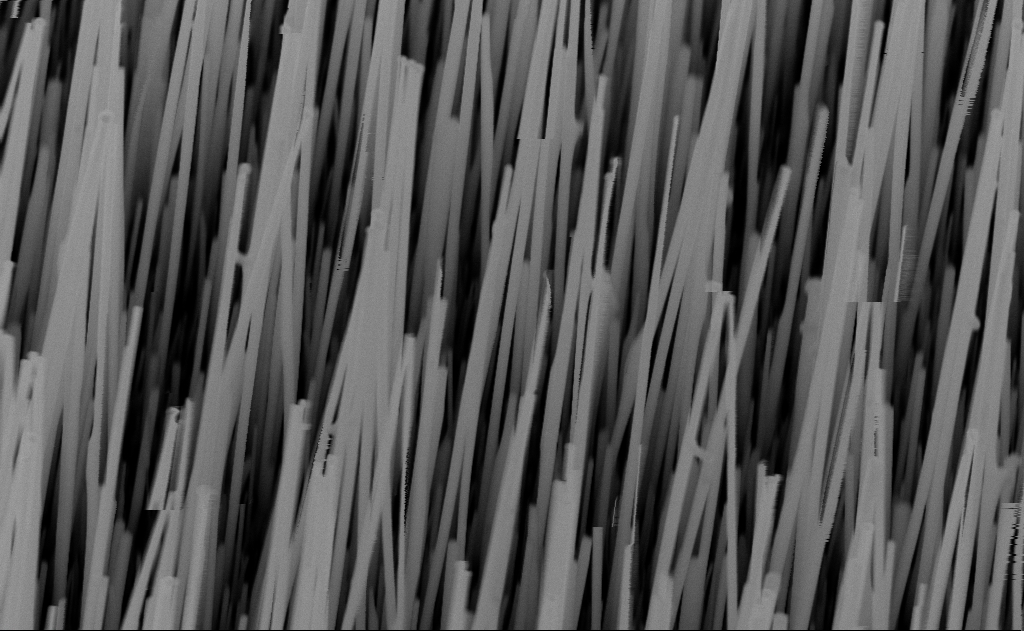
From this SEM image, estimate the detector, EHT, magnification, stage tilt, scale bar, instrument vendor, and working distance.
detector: InLens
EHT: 10 kV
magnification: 40 K X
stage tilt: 30°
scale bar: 1000 nm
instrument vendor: Zeiss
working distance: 8 mm